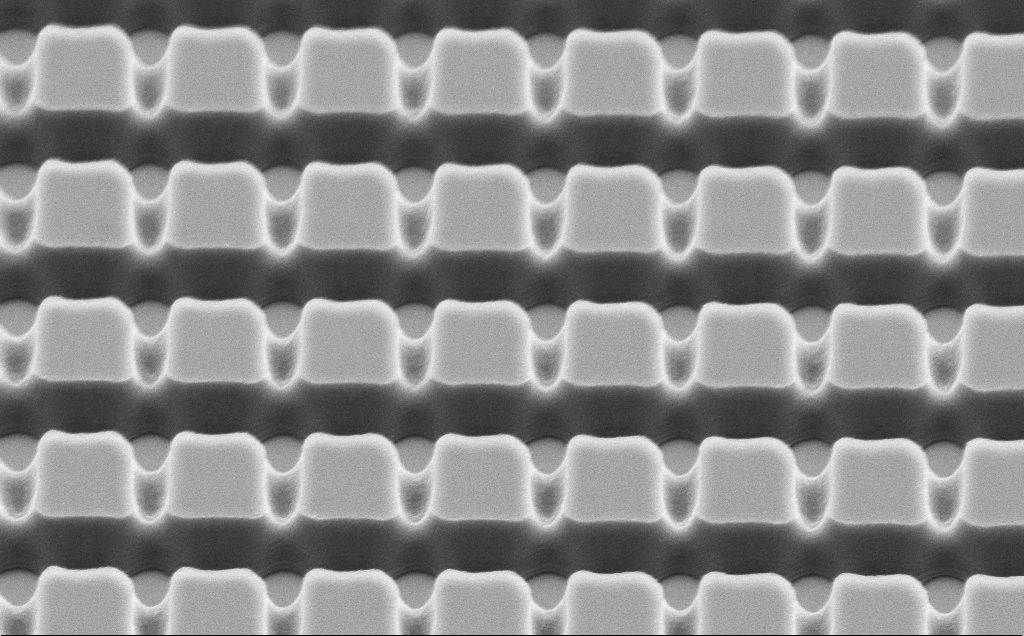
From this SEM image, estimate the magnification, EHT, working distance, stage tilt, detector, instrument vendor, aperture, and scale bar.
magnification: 12.11 K X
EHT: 5 kV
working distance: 10 mm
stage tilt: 45°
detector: SE2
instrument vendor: Zeiss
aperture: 30 µm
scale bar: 2000 nm